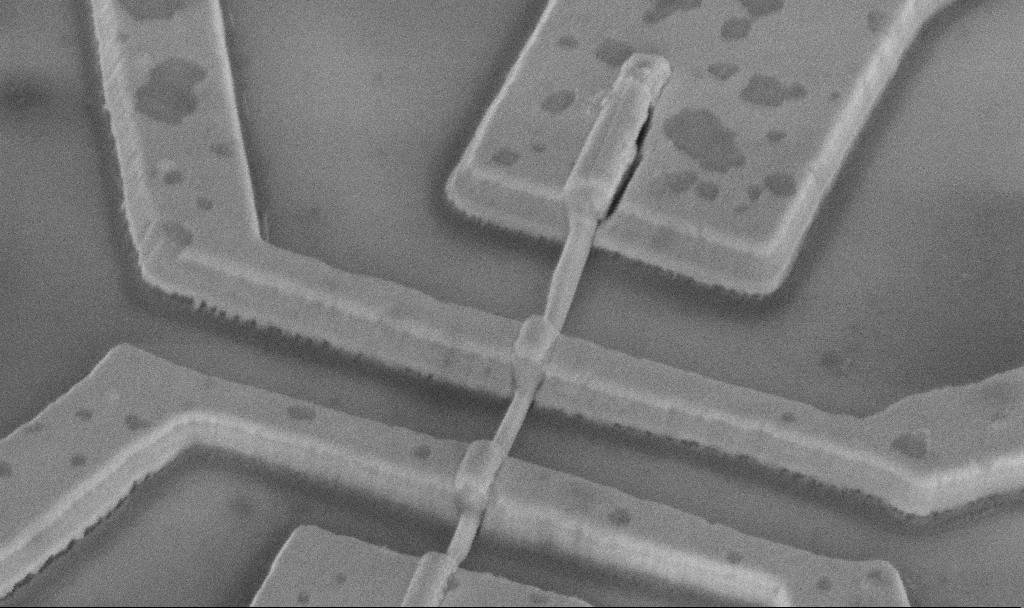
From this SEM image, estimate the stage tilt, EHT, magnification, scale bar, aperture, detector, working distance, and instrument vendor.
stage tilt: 45°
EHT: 5 kV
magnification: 60 K X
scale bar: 1000 nm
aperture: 30 µm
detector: InLens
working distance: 14.2 mm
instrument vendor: Zeiss